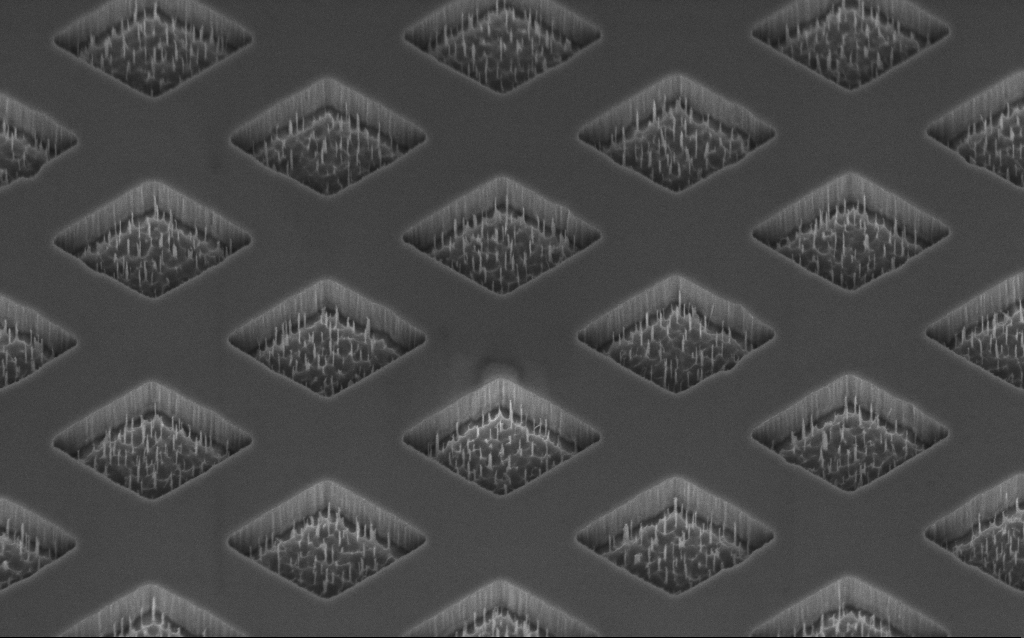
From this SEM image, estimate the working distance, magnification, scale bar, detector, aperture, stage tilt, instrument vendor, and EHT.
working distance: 8 mm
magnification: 25.47 K X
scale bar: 1000 nm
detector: InLens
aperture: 30 µm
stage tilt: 45°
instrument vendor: Zeiss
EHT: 3 kV